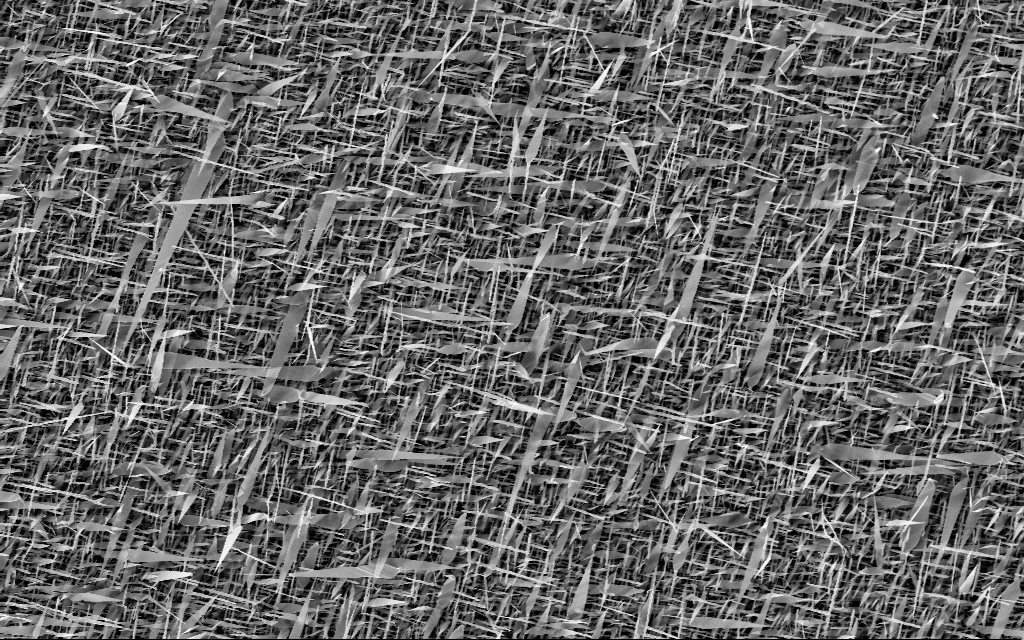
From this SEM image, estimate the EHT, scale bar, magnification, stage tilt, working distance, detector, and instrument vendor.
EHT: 10 kV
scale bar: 10000 nm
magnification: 5 K X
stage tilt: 0°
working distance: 7 mm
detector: InLens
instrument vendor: Zeiss